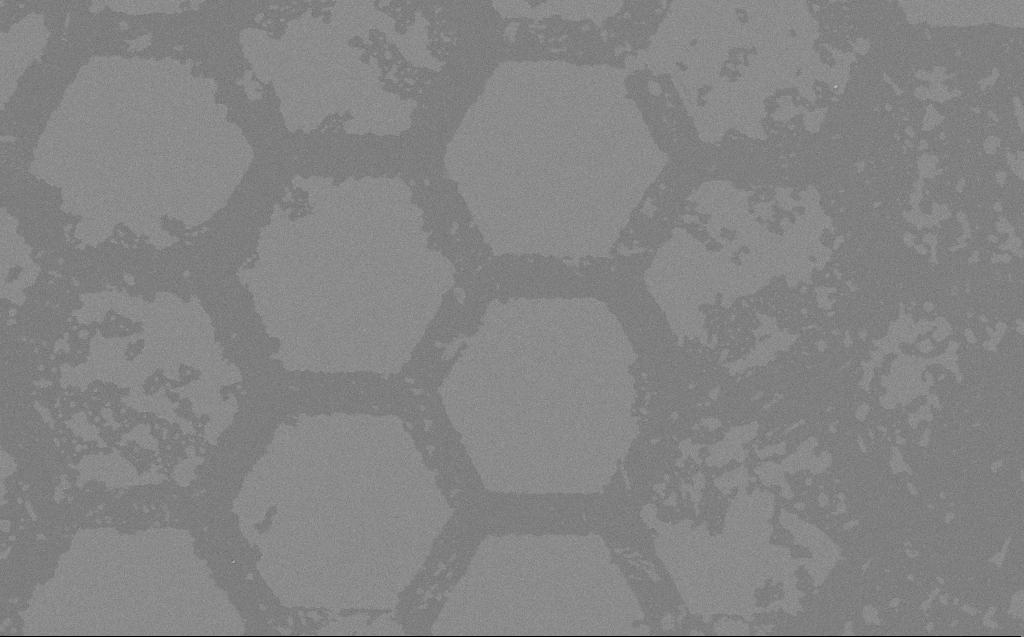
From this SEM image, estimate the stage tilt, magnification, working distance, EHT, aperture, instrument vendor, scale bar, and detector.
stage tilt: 0°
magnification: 1.46 K X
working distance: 5 mm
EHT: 3 kV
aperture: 30 µm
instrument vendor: Zeiss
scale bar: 20000 nm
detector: SE2